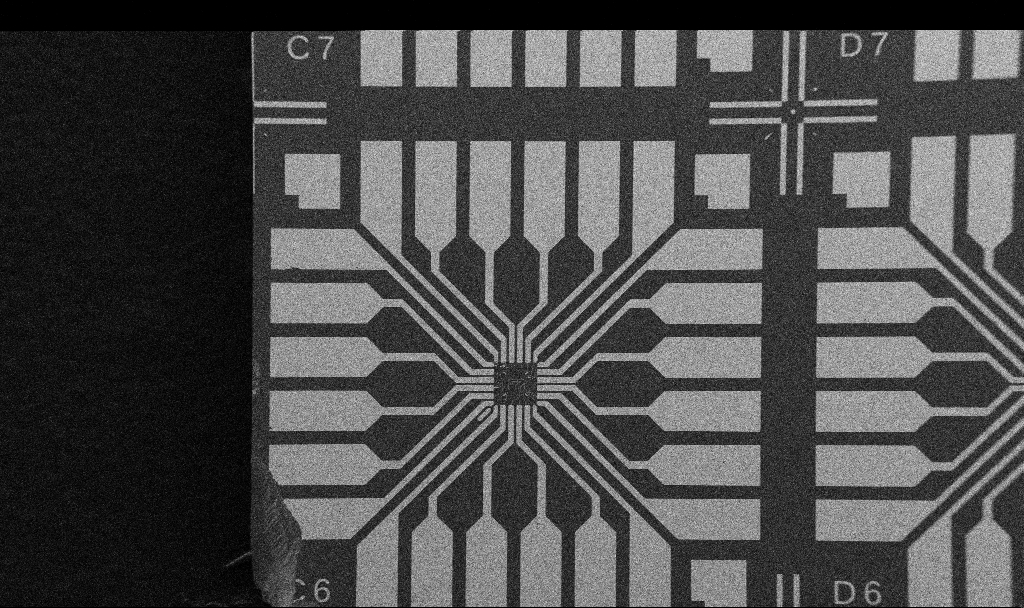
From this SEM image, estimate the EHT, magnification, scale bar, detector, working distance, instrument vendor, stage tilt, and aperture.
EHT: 5 kV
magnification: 0.1 K X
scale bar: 200000 nm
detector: SE2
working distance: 10.7 mm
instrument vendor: Zeiss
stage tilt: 0°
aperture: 30 µm